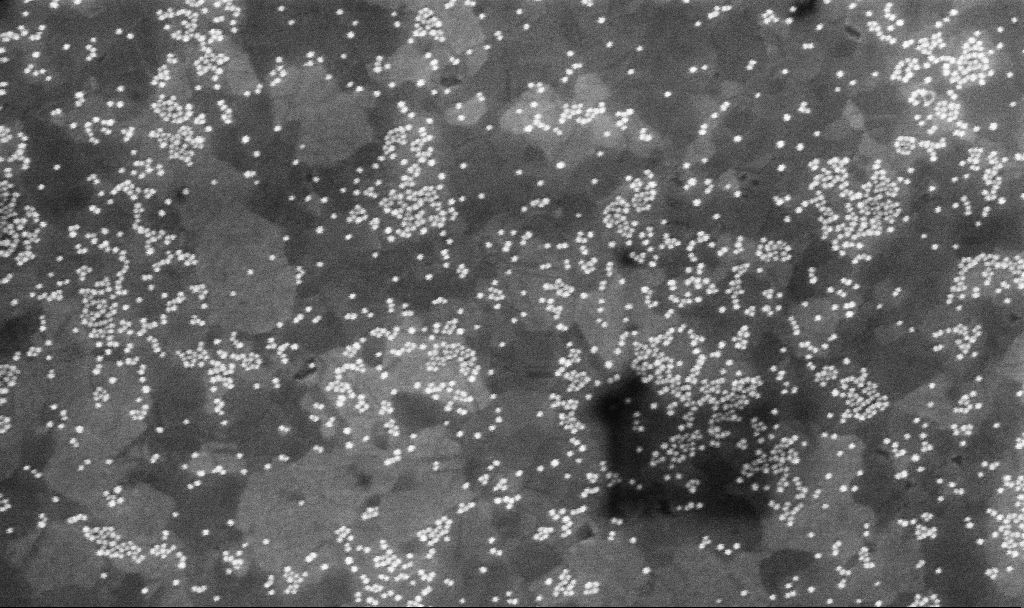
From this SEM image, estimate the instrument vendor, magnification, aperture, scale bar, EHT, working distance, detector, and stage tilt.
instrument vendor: Zeiss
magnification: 113.29 K X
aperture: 30 µm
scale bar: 200 nm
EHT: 5 kV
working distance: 7 mm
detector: InLens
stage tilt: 0°